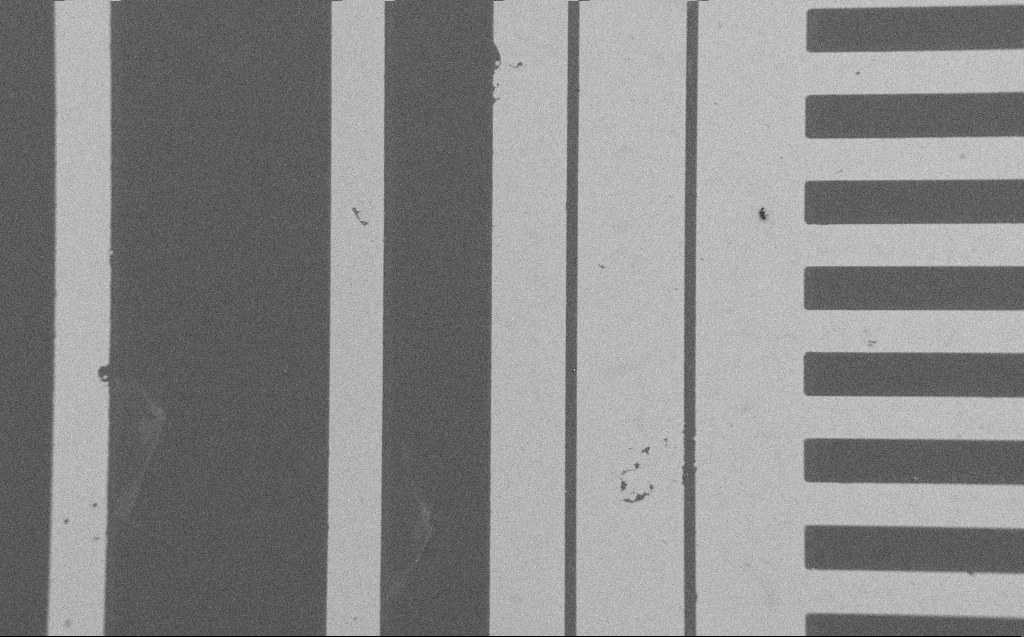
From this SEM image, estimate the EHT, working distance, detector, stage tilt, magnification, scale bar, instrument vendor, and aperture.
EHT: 1.2 kV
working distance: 6 mm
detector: SE2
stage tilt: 0°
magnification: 0.393 K X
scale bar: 100000 nm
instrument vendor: Zeiss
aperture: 30 µm